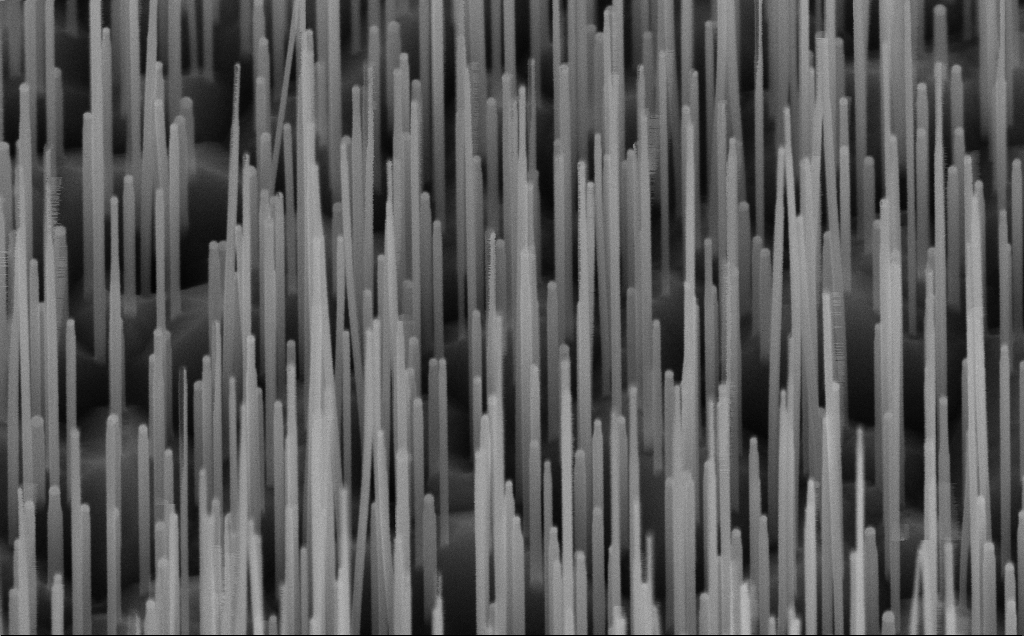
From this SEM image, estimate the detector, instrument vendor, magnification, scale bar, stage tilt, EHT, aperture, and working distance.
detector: InLens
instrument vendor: Zeiss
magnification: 100 K X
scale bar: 200 nm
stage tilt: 45°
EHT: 10 kV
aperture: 30 µm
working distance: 7 mm